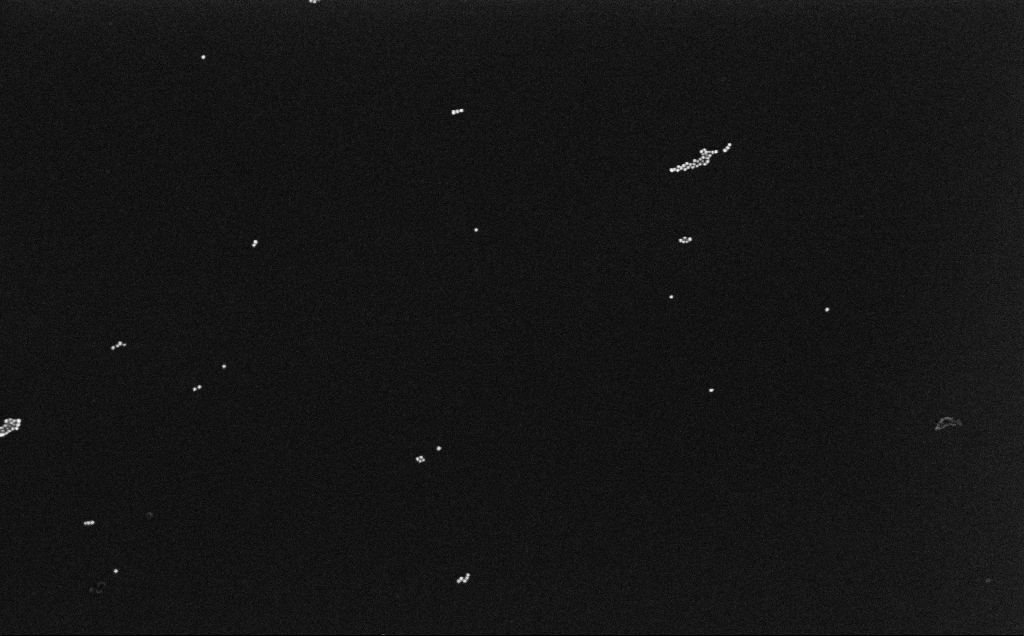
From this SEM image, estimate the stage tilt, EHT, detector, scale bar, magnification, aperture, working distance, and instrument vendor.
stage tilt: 0°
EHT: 10 kV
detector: InLens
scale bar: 200 nm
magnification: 100 K X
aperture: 30 µm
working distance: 6.6 mm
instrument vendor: Zeiss